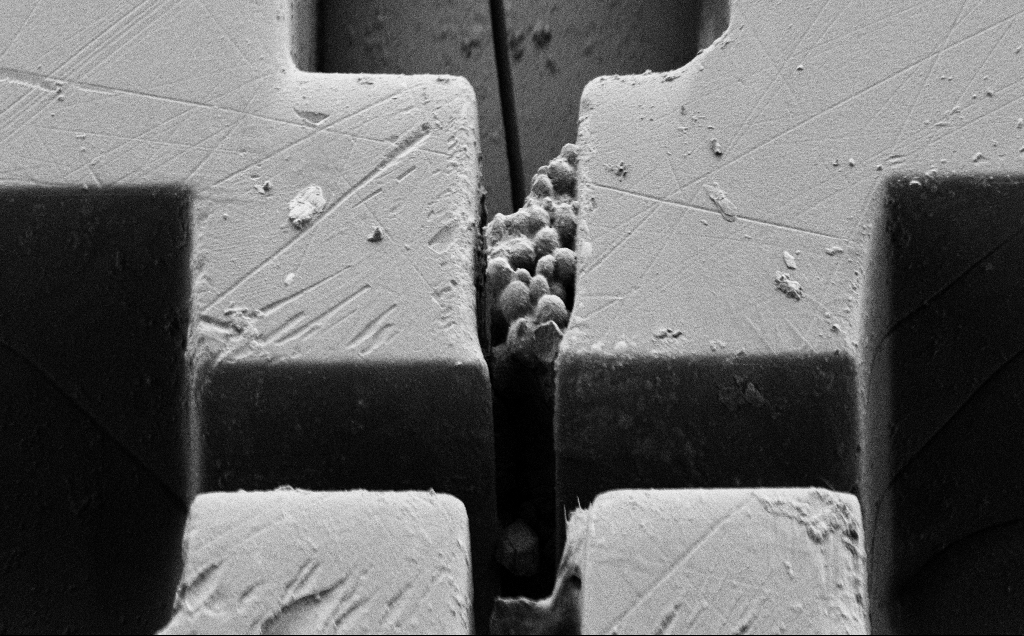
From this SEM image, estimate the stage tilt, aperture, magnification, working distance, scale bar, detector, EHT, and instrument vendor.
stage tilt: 45°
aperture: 30 µm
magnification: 3.14 K X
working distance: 5 mm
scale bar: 20000 nm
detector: SE2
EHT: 1 kV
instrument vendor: Zeiss